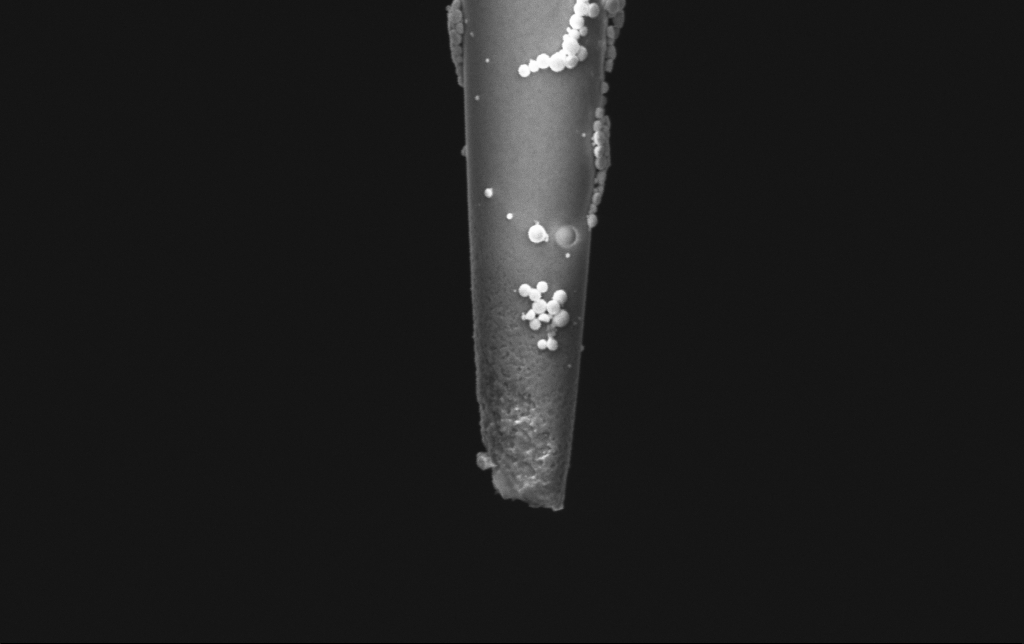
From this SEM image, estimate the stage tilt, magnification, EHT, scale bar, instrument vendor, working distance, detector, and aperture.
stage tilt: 0°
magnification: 50 K X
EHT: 2 kV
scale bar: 1000 nm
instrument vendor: Zeiss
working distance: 5.9 mm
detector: InLens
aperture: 30 µm